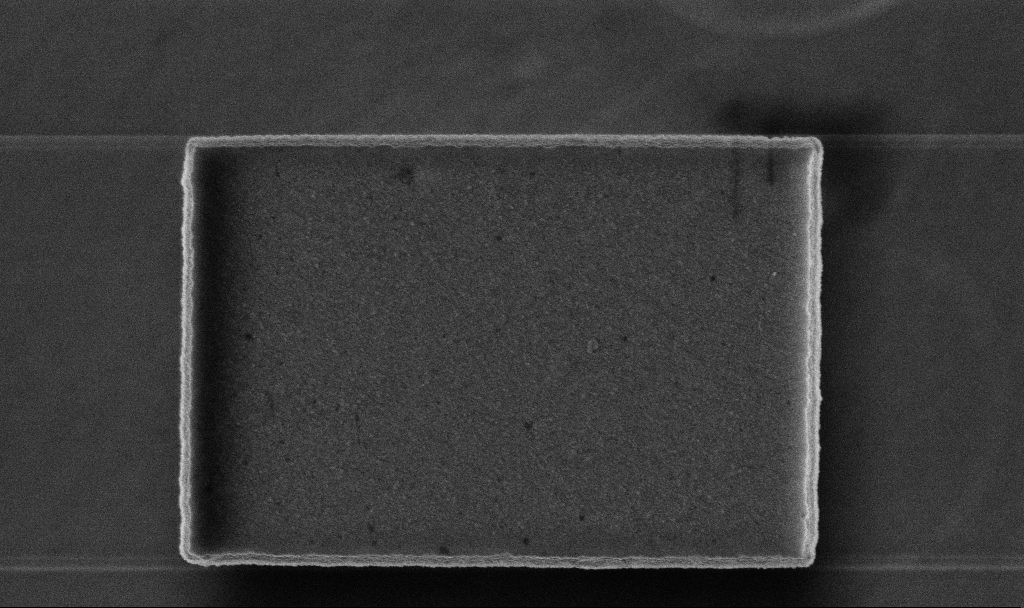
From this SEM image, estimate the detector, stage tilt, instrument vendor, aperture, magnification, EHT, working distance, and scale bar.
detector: InLens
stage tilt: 0°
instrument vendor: Zeiss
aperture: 30 µm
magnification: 52.34 K X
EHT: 5 kV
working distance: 3.2 mm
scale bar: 1000 nm